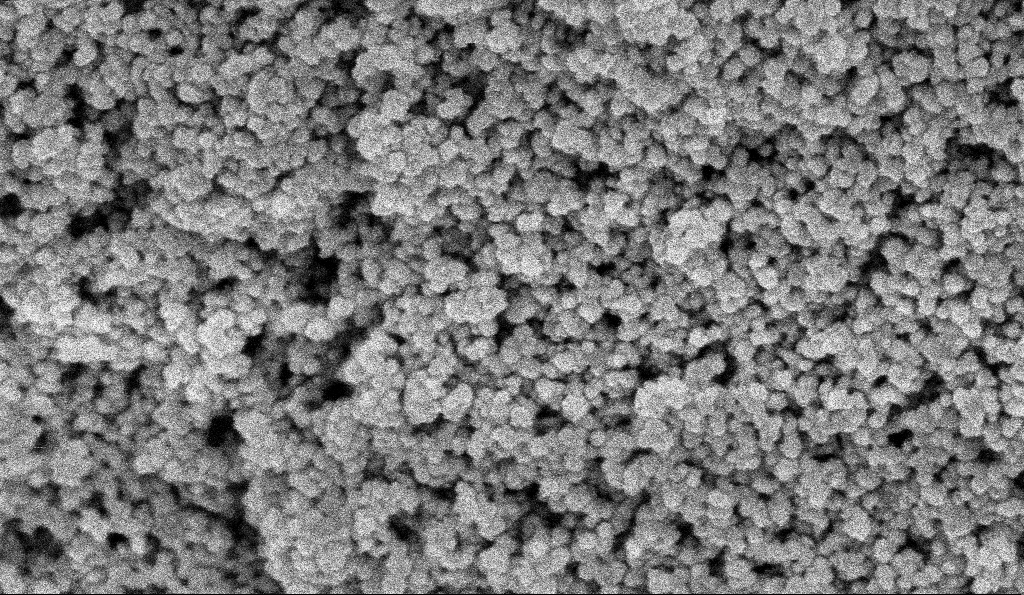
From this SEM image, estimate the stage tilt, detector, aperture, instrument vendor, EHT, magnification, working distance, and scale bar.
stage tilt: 0°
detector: InLens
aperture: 30 µm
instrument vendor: Zeiss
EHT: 5 kV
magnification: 135 K X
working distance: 6 mm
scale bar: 100 nm